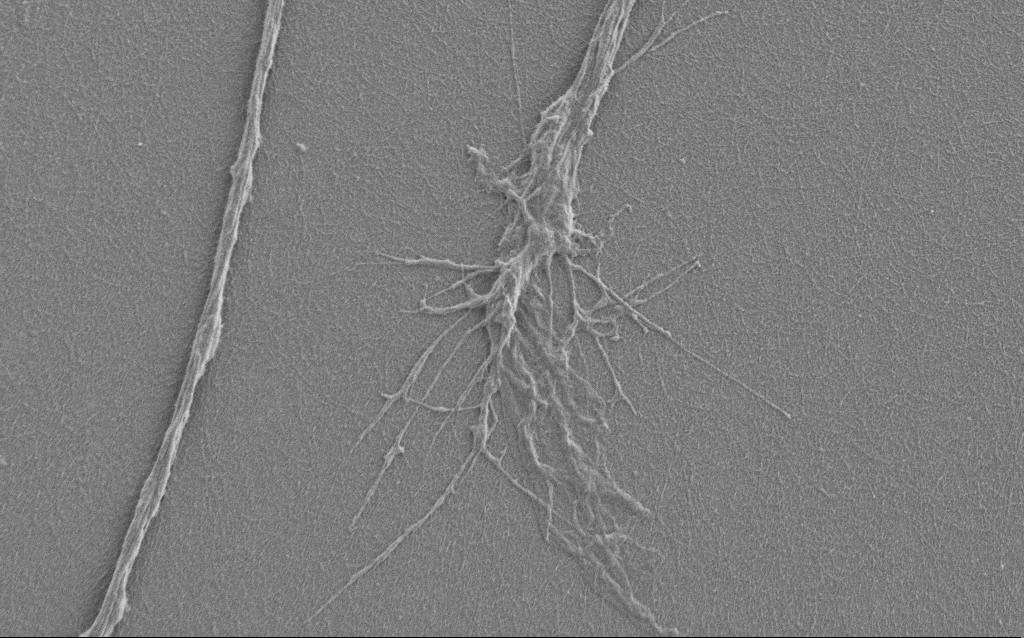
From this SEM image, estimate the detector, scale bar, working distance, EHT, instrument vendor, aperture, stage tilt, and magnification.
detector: SE2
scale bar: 2000 nm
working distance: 6 mm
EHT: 1 kV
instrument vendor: Zeiss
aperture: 30 µm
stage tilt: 0°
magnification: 7.5 K X